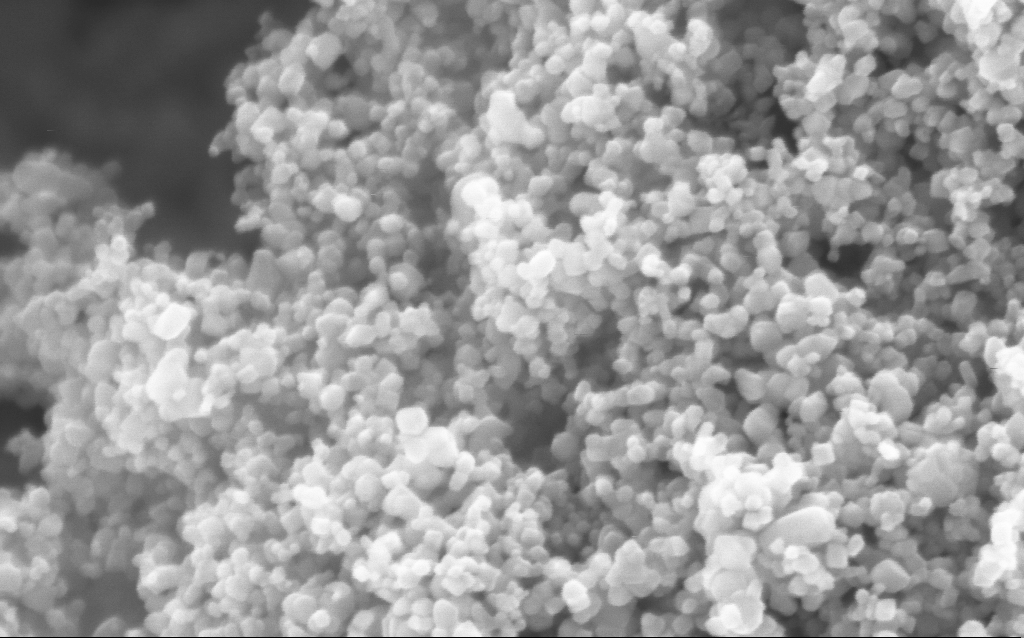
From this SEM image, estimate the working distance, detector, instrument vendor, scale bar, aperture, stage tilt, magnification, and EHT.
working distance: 4.4 mm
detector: InLens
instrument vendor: Zeiss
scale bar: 200 nm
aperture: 30 µm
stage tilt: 0°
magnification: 294.51 K X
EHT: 5 kV